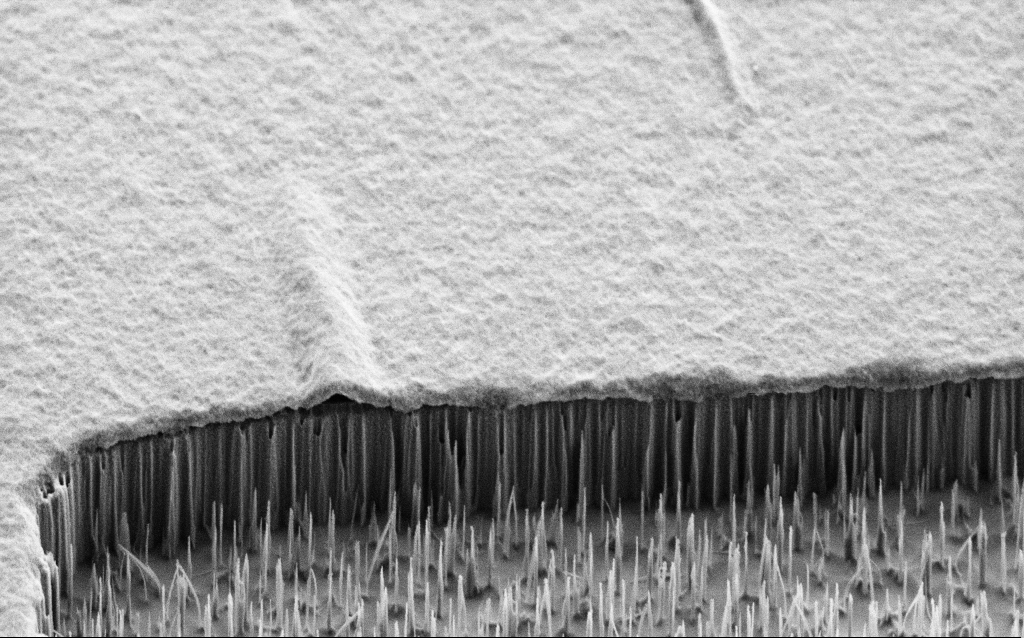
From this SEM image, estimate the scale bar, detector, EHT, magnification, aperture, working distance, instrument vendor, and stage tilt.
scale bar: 1000 nm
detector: SE2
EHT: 2 kV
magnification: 17.27 K X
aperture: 30 µm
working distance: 7 mm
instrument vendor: Zeiss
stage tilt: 45°